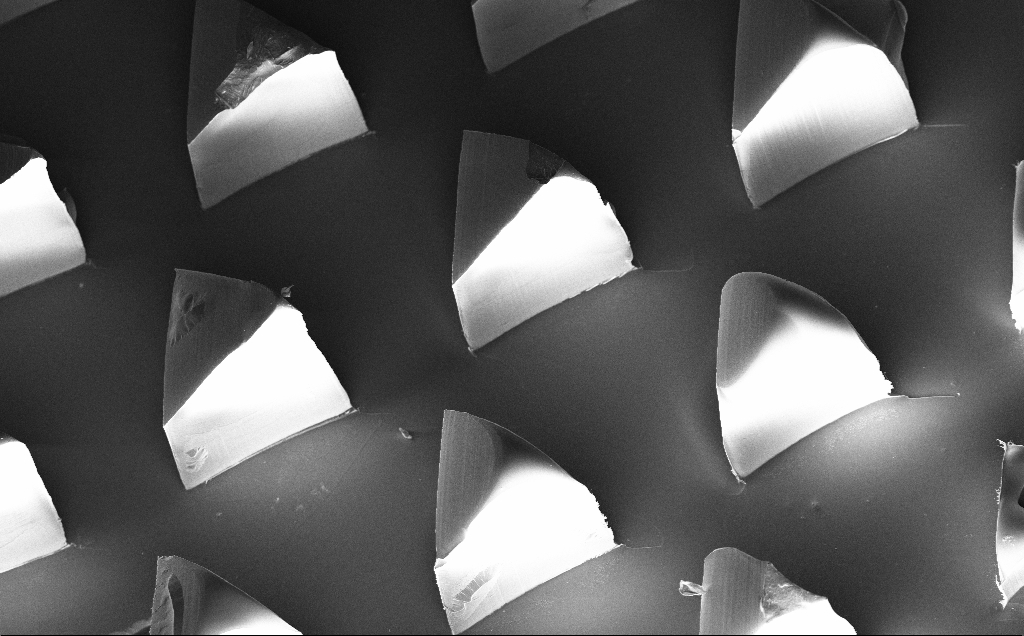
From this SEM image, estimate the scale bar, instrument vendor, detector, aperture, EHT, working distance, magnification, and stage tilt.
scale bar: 100000 nm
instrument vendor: Zeiss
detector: InLens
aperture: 30 µm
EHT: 10 kV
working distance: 10 mm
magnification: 0.258 K X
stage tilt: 20°